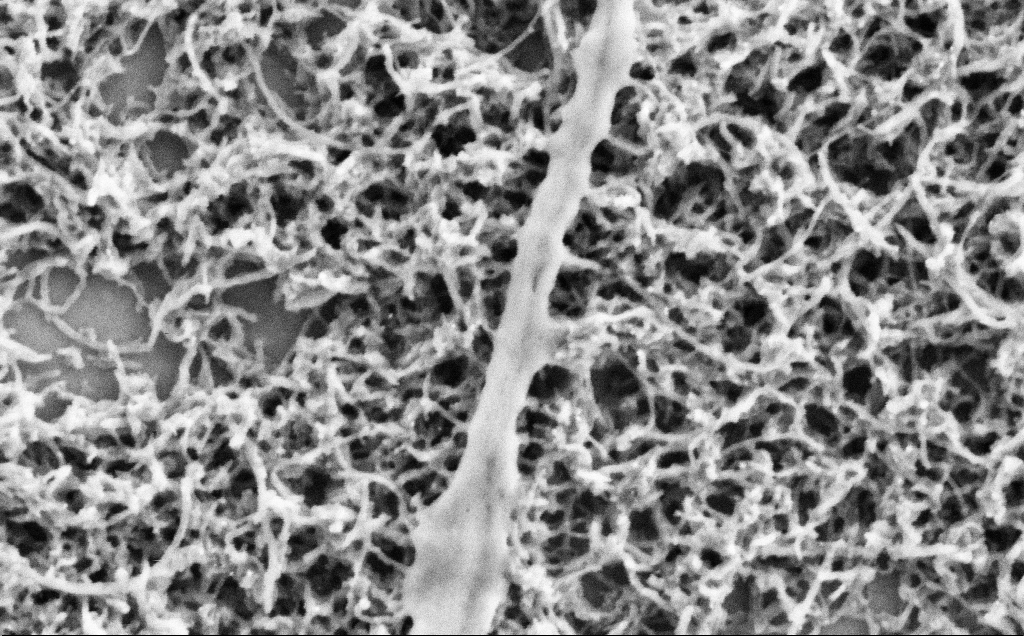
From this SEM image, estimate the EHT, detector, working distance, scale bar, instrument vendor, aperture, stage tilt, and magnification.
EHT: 2 kV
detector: SE2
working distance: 7.1 mm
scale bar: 200 nm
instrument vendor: Zeiss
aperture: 30 µm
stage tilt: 0°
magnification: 80 K X